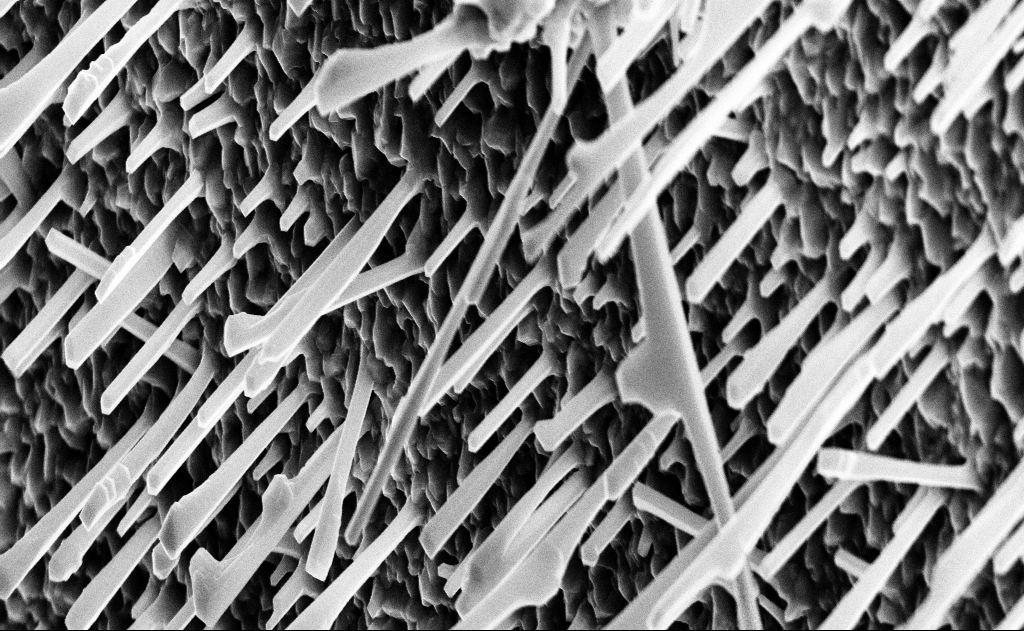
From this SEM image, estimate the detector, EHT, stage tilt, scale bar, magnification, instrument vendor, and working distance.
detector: InLens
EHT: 10 kV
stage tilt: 0°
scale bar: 1000 nm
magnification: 20 K X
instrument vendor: Zeiss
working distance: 15 mm